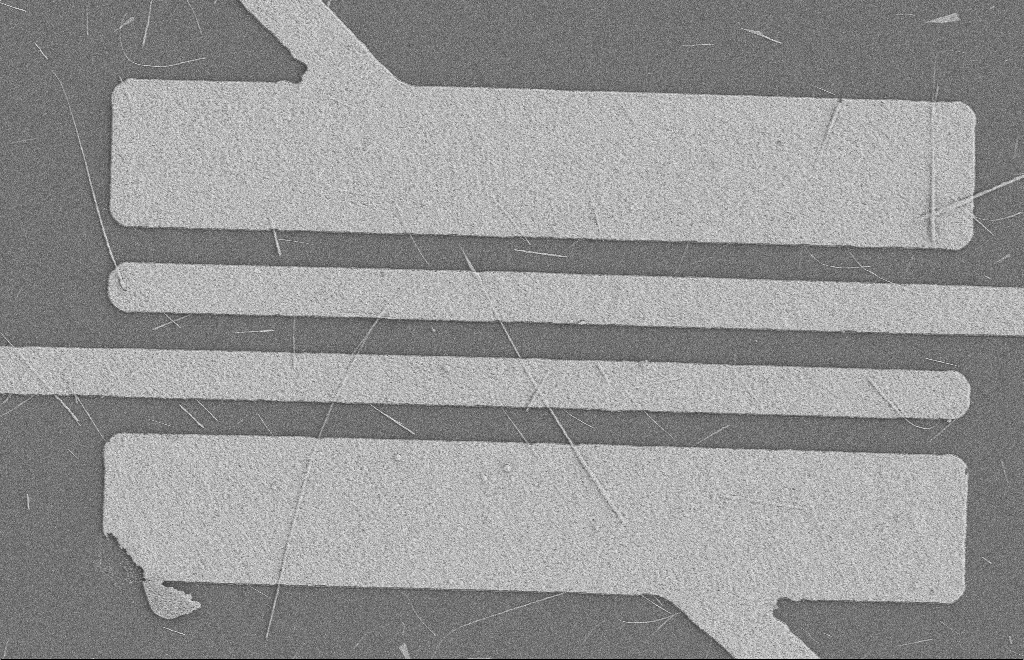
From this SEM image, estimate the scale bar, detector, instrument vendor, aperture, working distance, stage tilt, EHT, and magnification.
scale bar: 2000 nm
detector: SE2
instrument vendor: Zeiss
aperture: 20 µm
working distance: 8 mm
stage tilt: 0°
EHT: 2 kV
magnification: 5.2 K X